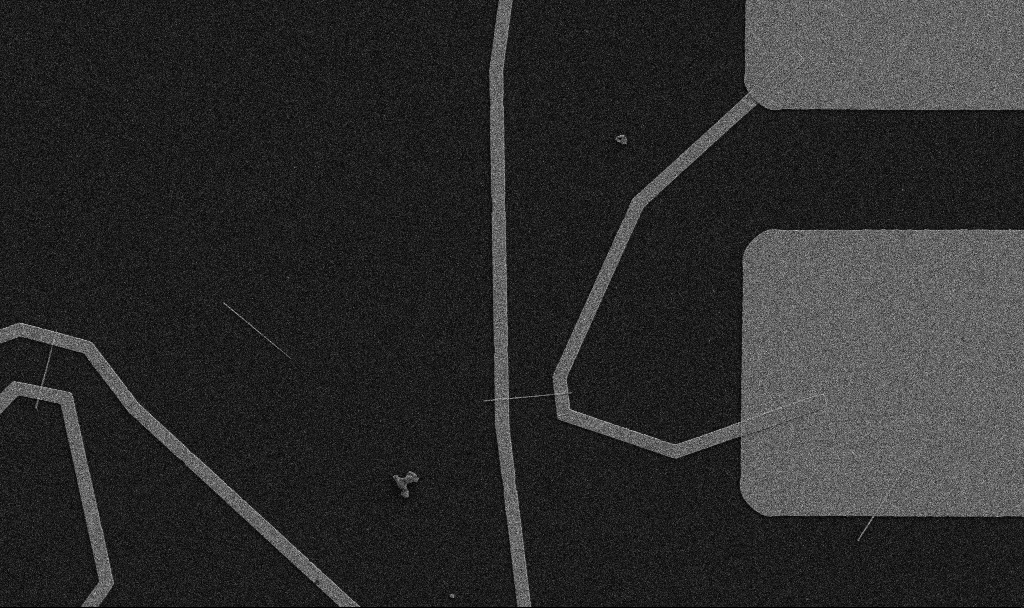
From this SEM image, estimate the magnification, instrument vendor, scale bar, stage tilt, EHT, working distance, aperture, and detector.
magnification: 5 K X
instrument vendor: Zeiss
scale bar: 10000 nm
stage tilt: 0°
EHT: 5 kV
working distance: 10.7 mm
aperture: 30 µm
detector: SE2